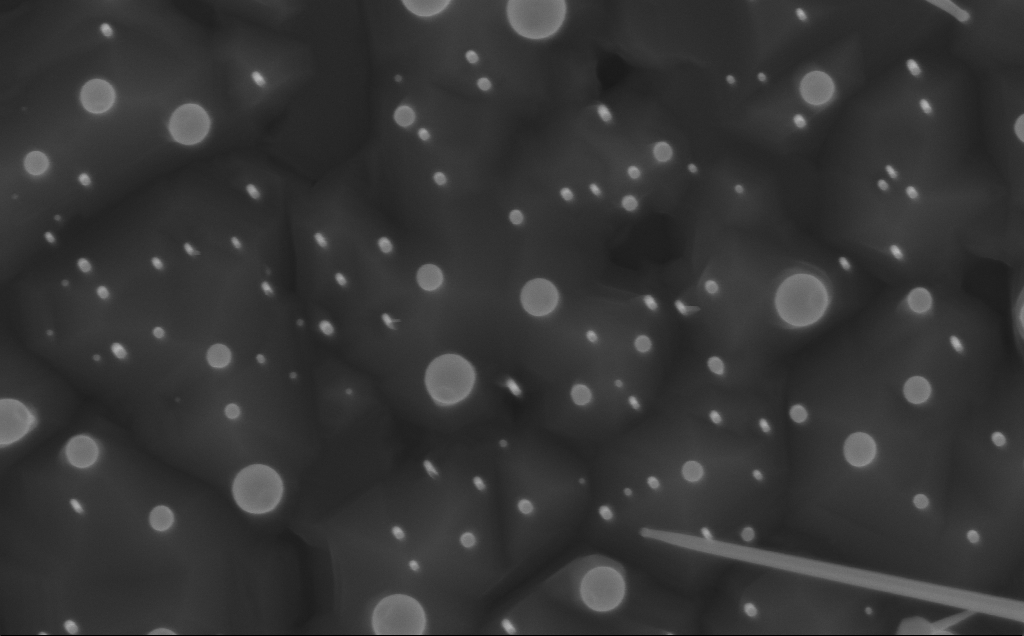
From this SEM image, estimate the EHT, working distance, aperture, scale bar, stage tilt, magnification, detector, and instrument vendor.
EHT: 10 kV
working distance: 3 mm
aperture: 30 µm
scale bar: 200 nm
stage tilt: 0°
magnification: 82.37 K X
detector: InLens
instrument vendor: Zeiss